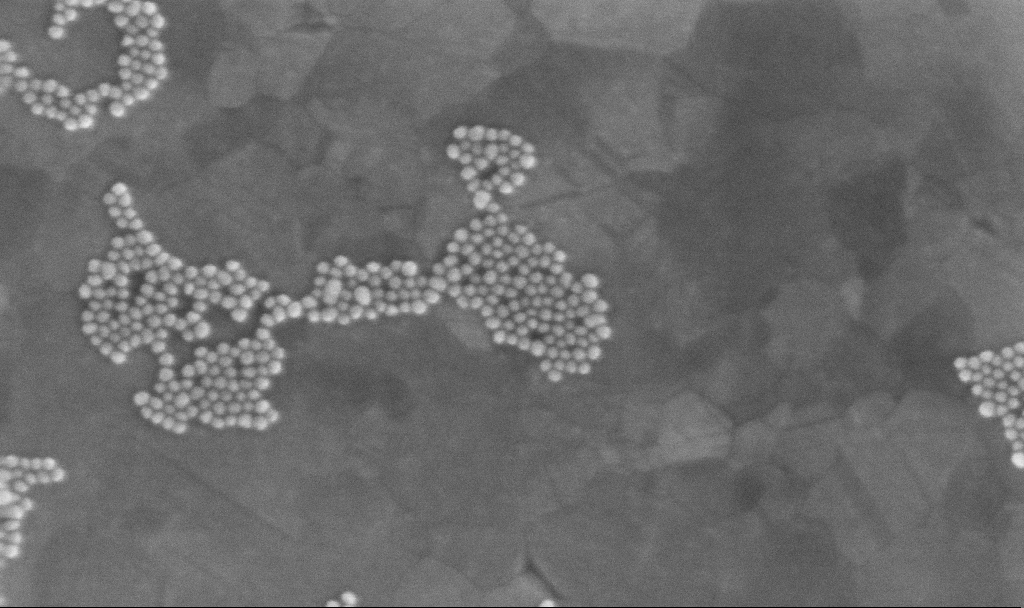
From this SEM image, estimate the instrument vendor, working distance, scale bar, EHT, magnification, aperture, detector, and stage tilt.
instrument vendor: Zeiss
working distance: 3.4 mm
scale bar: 100 nm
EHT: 10 kV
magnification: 300 K X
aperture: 30 µm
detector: InLens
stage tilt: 0°